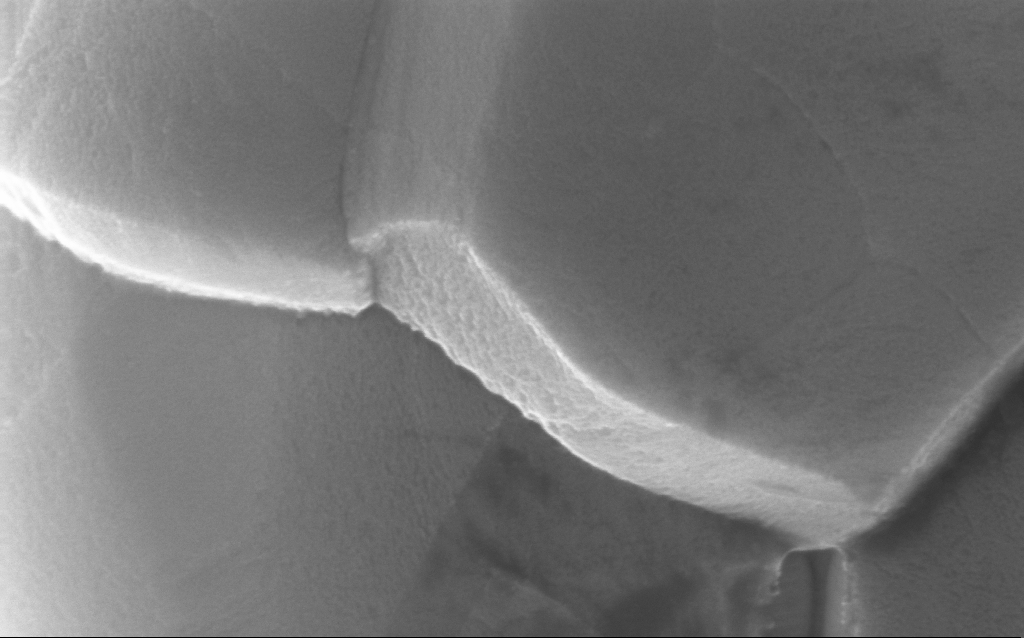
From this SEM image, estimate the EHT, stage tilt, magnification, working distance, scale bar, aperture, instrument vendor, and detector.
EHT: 10 kV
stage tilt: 0°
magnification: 279.01 K X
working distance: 2 mm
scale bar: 200 nm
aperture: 30 µm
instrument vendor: Zeiss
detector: InLens